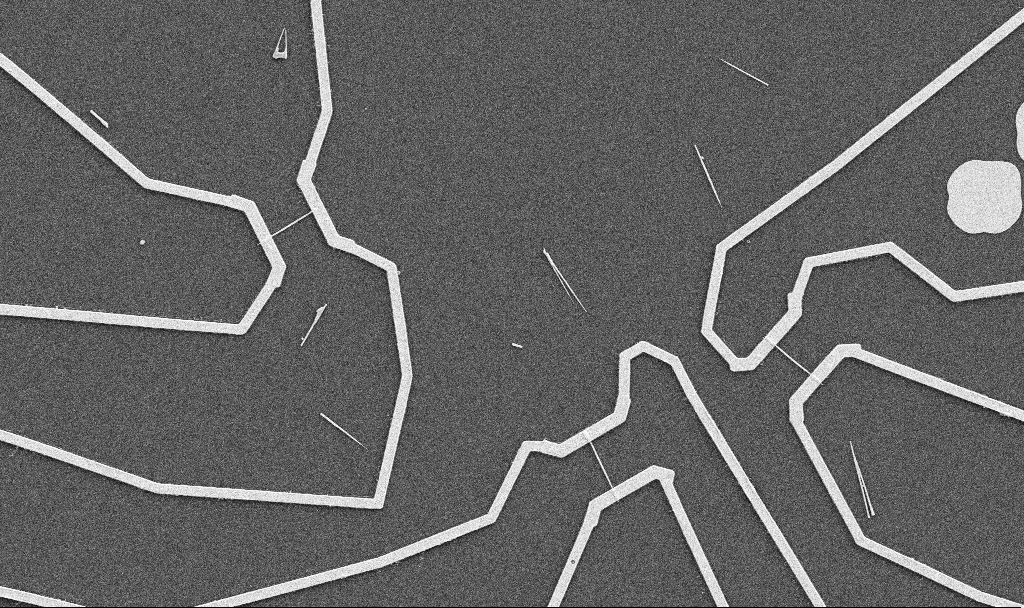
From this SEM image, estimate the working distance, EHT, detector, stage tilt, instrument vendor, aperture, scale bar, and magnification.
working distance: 10.7 mm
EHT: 5 kV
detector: SE2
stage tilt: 0°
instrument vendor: Zeiss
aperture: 30 µm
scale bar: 10000 nm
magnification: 5 K X